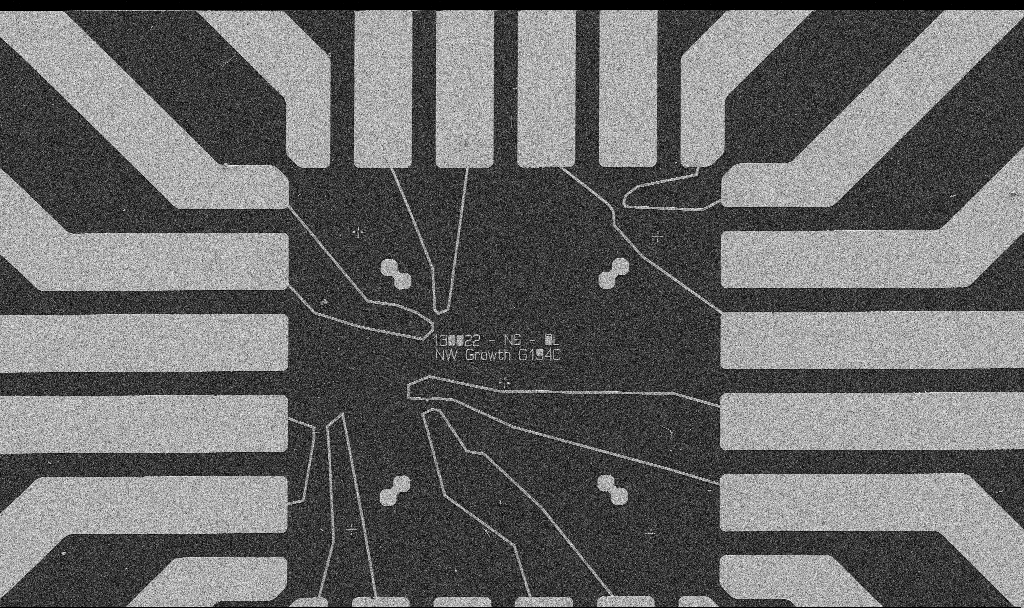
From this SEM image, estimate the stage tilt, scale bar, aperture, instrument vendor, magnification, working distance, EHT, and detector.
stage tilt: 0°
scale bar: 20000 nm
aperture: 30 µm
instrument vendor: Zeiss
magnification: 1 K X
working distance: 8.7 mm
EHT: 5 kV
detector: SE2